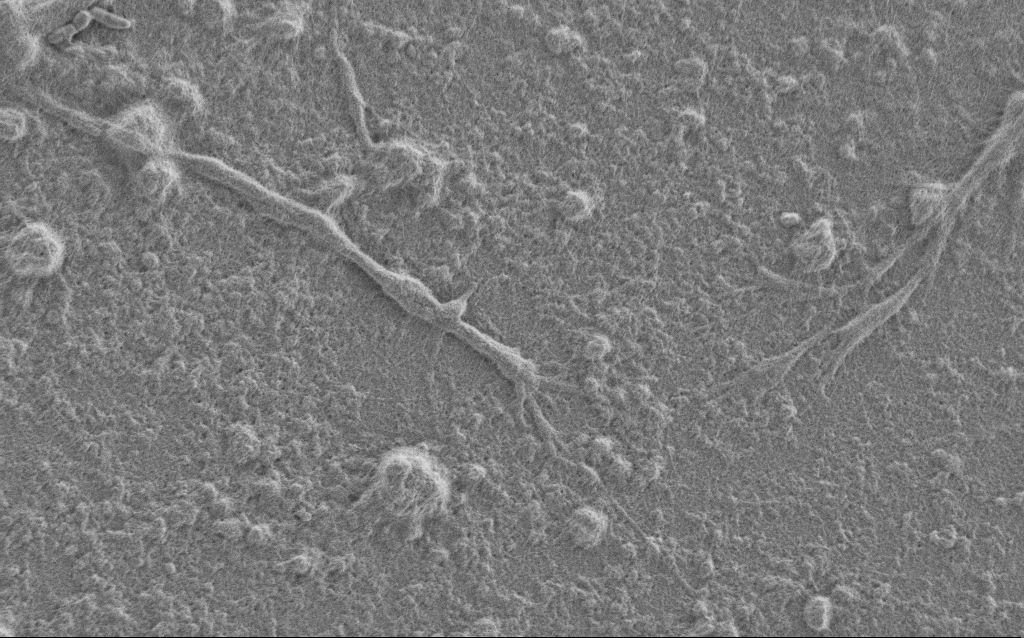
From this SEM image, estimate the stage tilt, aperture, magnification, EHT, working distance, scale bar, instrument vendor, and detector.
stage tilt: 0°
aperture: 30 µm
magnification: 7.5 K X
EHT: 1 kV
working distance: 6 mm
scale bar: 2000 nm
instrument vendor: Zeiss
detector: SE2